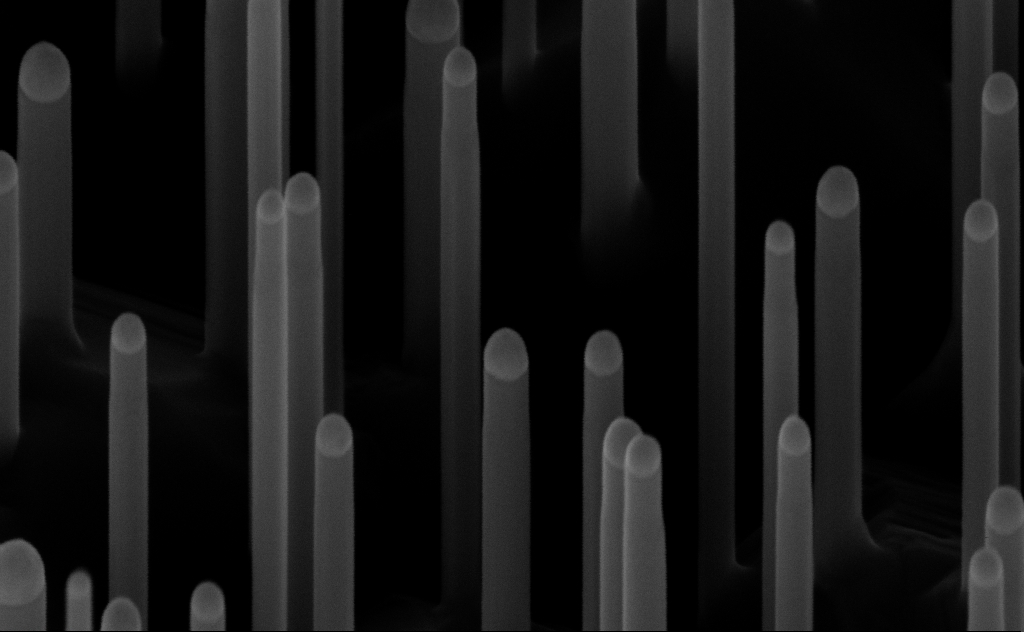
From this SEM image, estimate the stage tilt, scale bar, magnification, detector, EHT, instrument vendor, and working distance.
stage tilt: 45°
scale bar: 200 nm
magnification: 200 K X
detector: InLens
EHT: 10 kV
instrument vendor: Zeiss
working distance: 7 mm